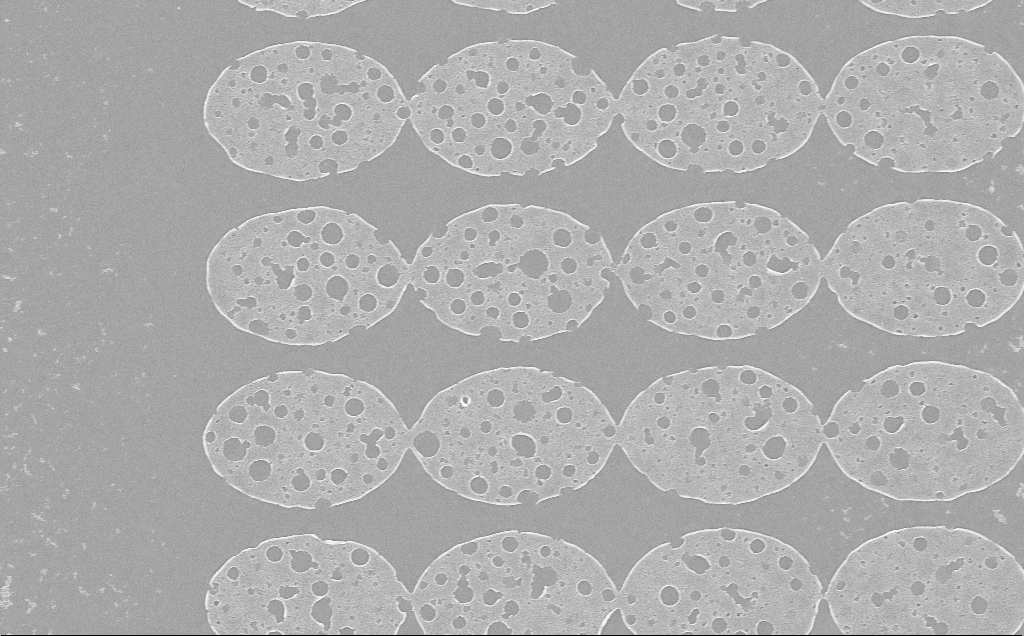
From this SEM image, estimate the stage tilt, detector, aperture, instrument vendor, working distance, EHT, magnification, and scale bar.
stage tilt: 0°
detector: InLens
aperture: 30 µm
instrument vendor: Zeiss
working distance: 6 mm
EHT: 5 kV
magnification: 7.64 K X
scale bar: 2000 nm